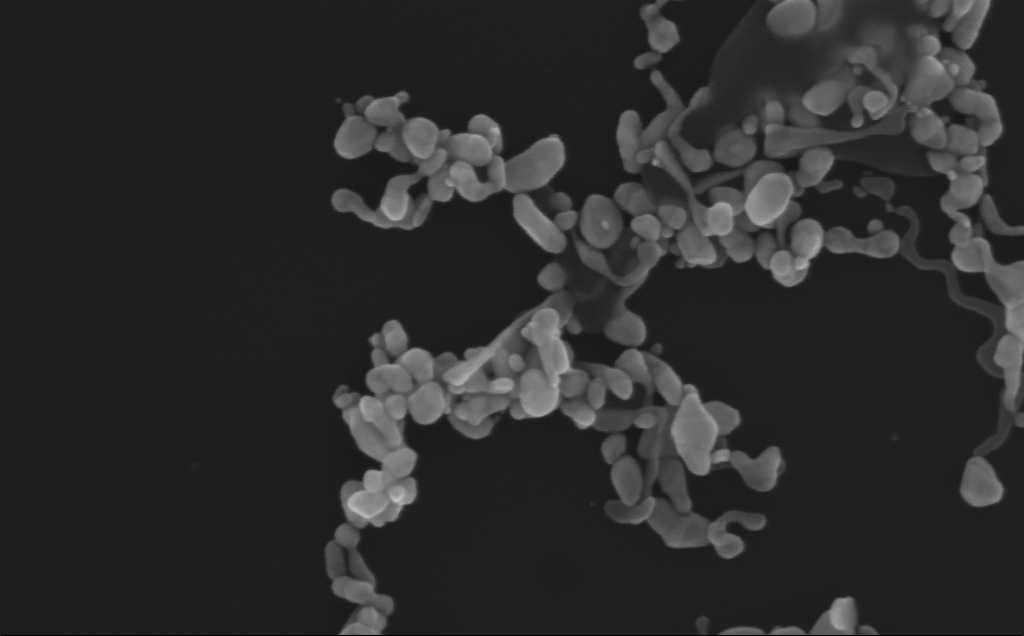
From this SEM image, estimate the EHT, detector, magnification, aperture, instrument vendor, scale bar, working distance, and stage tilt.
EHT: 10 kV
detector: InLens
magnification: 208.62 K X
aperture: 30 µm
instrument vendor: Zeiss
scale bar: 200 nm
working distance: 3 mm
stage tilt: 0°